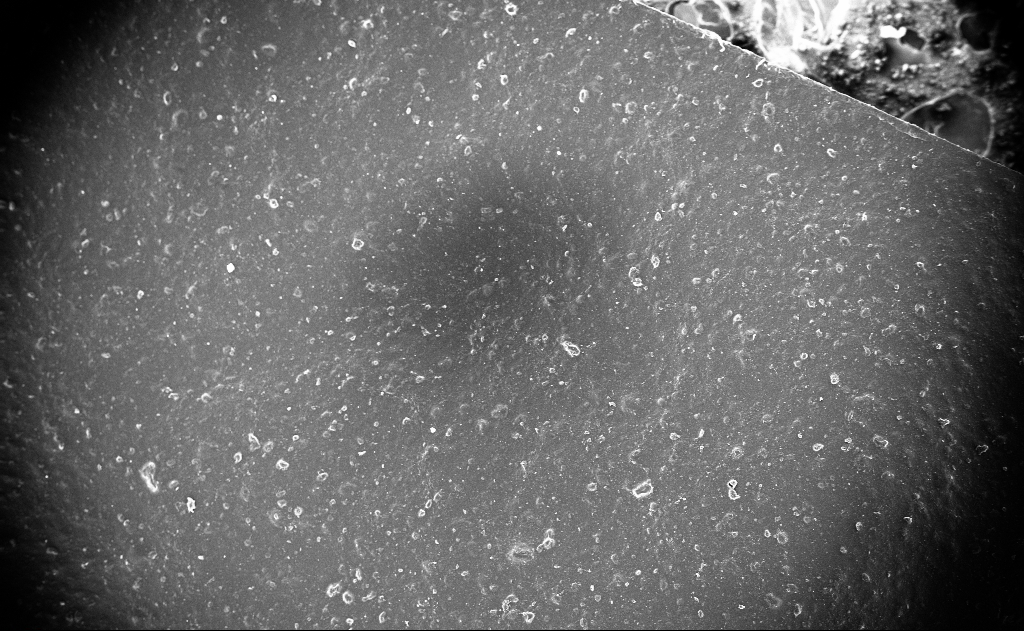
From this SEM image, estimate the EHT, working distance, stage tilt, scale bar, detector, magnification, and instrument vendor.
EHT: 10 kV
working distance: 5 mm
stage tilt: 0°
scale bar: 100000 nm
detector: InLens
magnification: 0.129 K X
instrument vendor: Zeiss